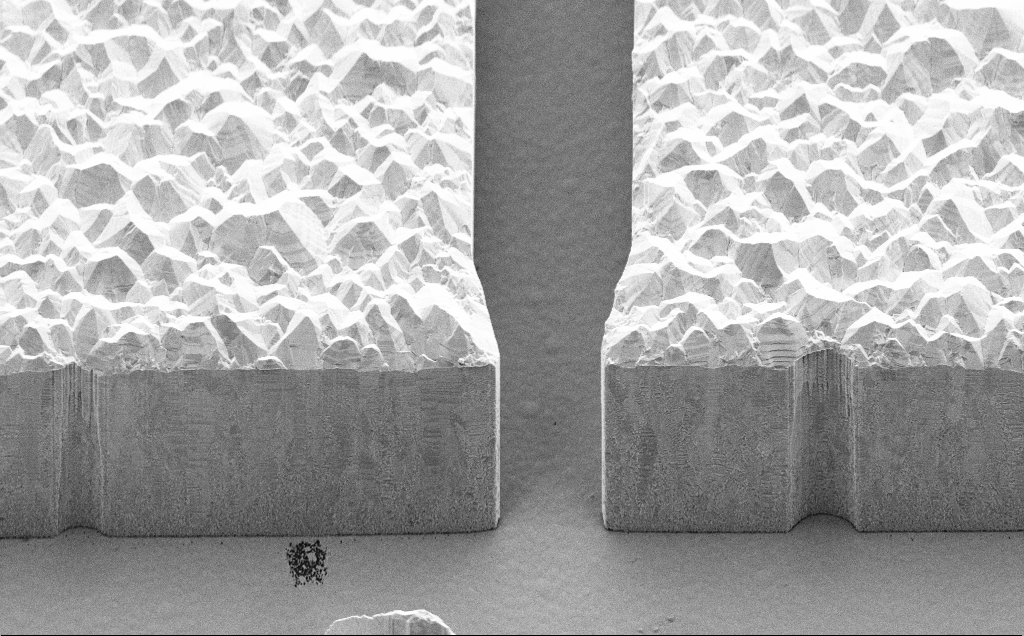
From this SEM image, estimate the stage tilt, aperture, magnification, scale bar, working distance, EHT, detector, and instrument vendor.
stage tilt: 45°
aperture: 30 µm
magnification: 5.74 K X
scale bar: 10000 nm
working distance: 10 mm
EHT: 5 kV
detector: SE2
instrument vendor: Zeiss